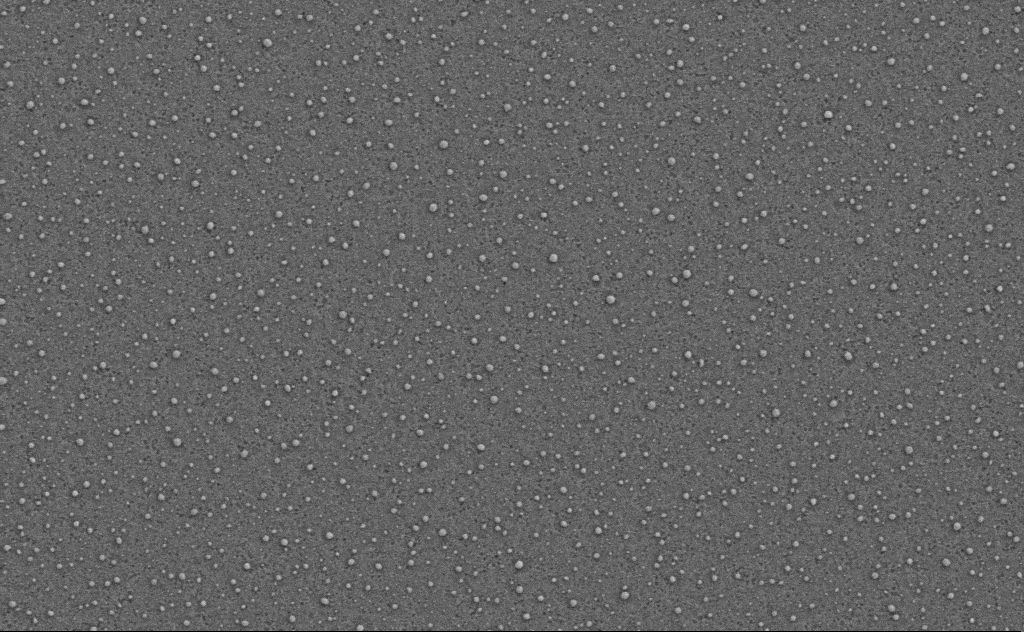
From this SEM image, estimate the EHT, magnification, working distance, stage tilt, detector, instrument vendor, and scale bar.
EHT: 3 kV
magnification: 40 K X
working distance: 4 mm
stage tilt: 0°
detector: SE2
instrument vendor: Zeiss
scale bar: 1000 nm